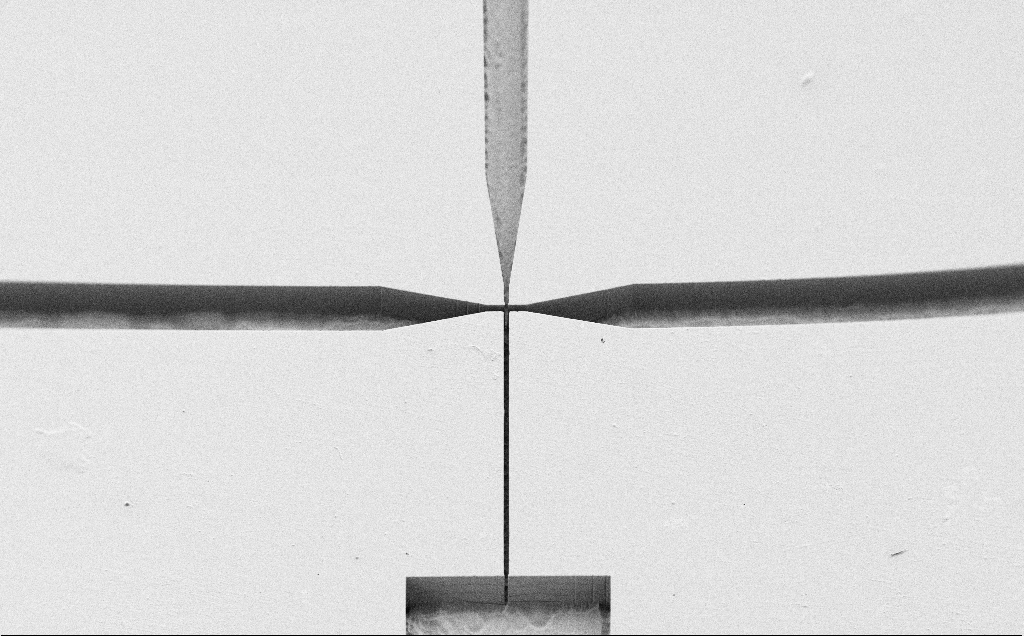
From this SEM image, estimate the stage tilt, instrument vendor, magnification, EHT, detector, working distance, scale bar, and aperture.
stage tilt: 45°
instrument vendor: Zeiss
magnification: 0.191 K X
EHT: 1.2 kV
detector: SE2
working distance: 5 mm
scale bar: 200000 nm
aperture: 30 µm